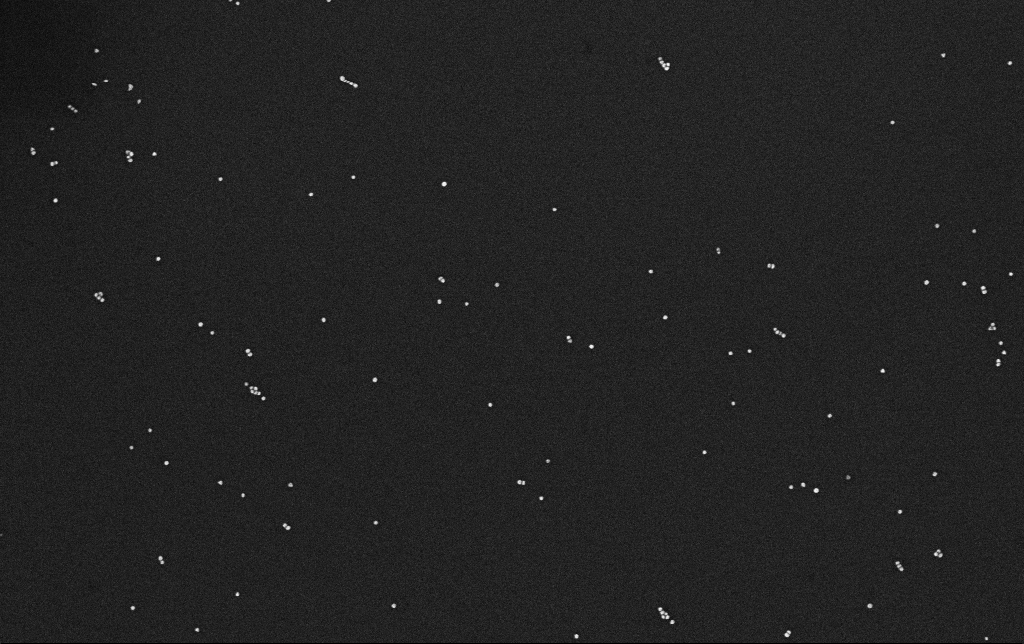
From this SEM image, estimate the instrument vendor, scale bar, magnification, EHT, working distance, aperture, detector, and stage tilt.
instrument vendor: Zeiss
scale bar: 200 nm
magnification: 100 K X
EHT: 10 kV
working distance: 3.1 mm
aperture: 30 µm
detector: InLens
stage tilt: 0°